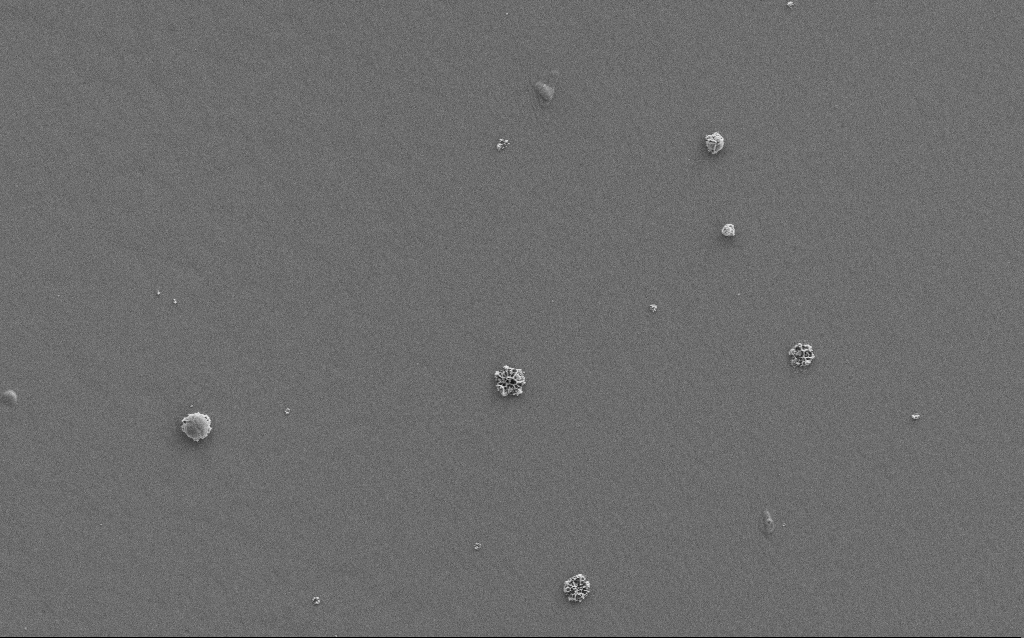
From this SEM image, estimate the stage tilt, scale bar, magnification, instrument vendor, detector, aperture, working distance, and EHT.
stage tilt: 0°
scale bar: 10000 nm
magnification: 3.76 K X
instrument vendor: Zeiss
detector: SE2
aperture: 30 µm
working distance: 2 mm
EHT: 10 kV